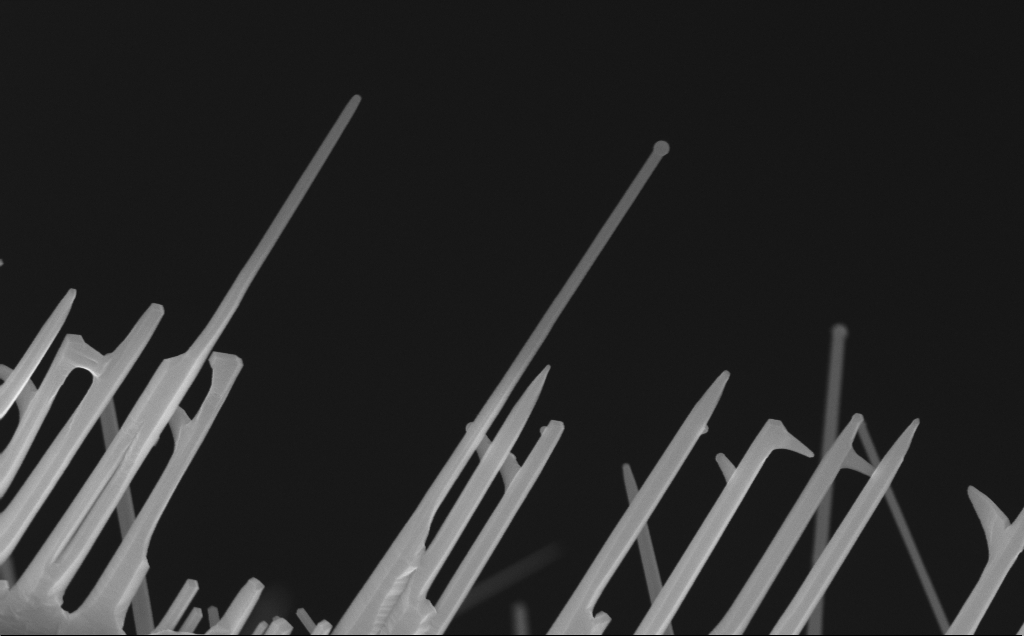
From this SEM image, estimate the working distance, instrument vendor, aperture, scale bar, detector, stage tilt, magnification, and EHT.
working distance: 7 mm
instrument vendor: Zeiss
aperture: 30 µm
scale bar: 1000 nm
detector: InLens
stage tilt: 0°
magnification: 47.7 K X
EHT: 10 kV